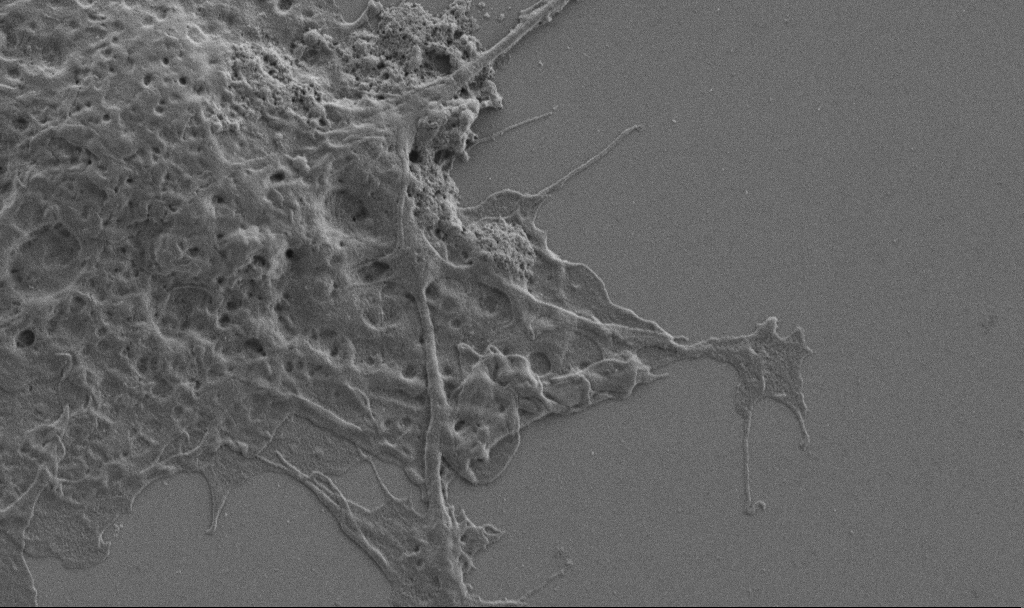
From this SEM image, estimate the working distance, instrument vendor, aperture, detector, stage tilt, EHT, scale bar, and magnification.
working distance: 6.9 mm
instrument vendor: Zeiss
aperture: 30 µm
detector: SE2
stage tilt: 0°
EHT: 1 kV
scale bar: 2000 nm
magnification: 10 K X